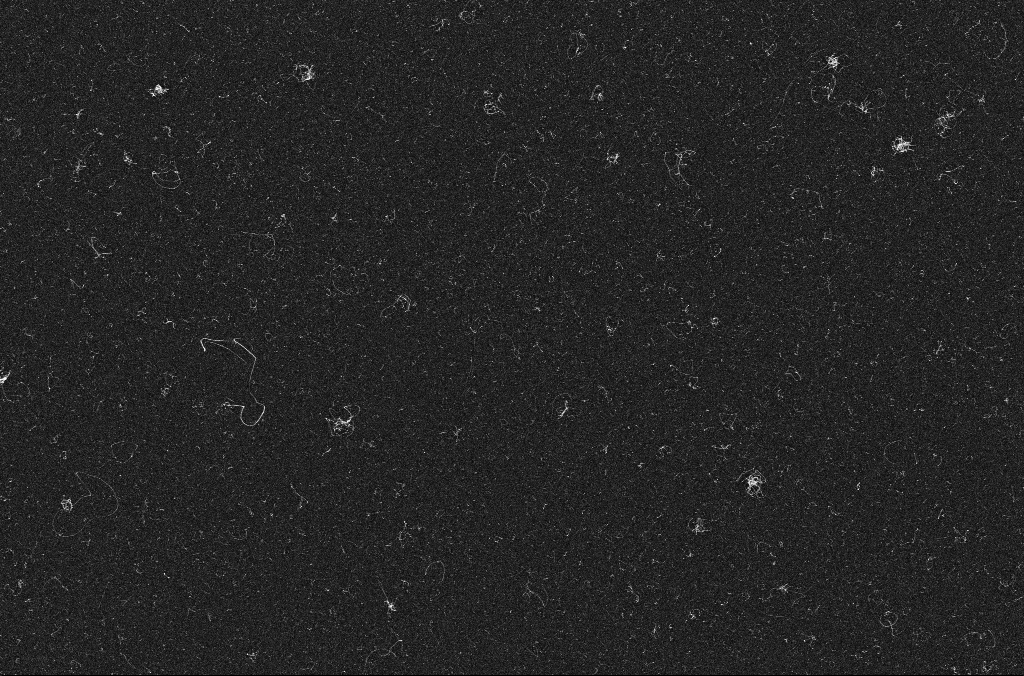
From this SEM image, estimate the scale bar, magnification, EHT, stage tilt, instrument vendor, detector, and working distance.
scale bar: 1000 nm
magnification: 20 K X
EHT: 10 kV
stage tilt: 0°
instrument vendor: Zeiss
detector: InLens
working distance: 3.3 mm